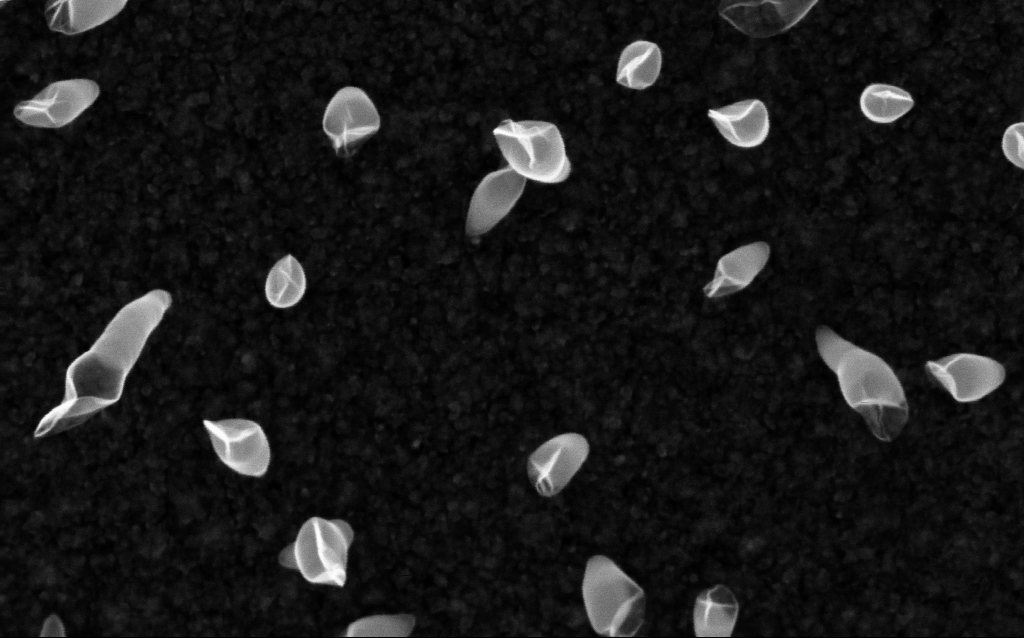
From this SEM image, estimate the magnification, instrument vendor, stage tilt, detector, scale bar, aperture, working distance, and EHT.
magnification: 200 K X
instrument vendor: Zeiss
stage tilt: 0°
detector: InLens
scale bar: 100 nm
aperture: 30 µm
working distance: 2.1 mm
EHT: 5 kV